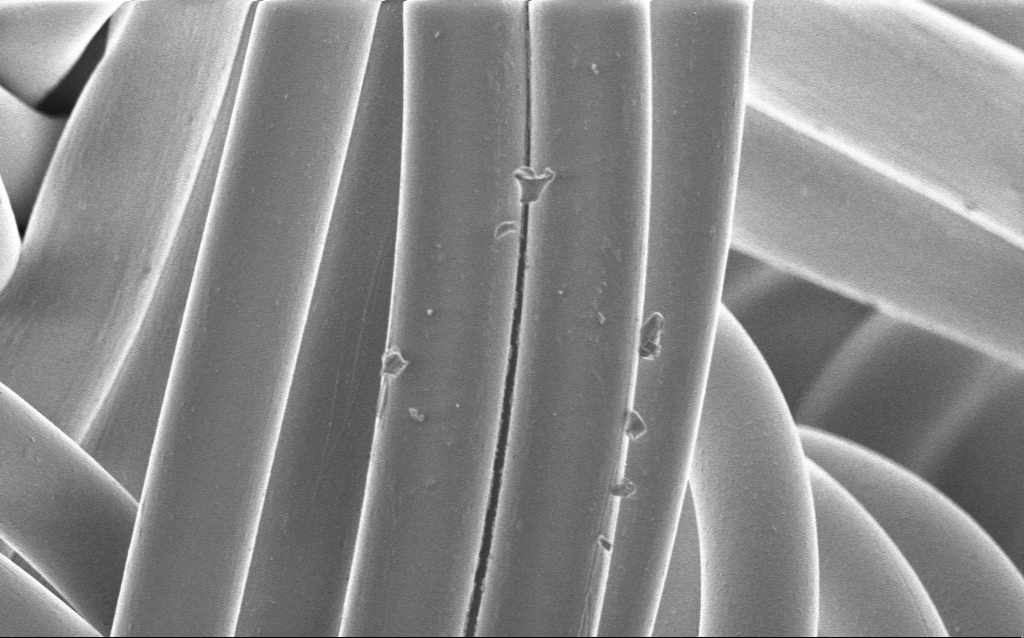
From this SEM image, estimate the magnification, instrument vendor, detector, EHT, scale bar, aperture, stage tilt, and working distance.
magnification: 1.87 K X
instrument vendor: Zeiss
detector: InLens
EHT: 1 kV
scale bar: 20000 nm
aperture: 30 µm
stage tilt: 0°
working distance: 4 mm